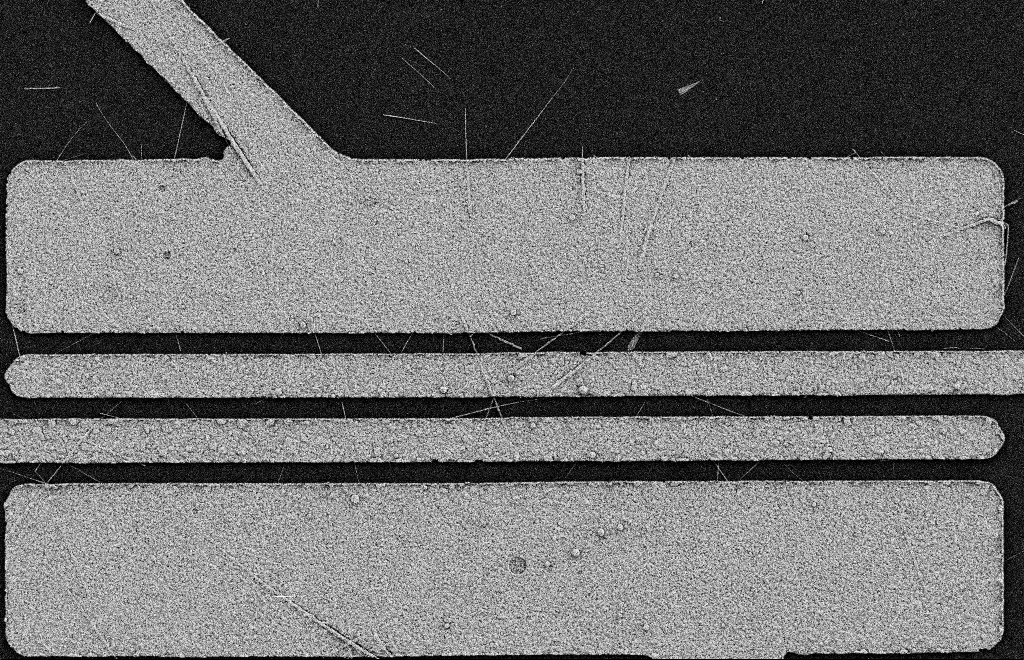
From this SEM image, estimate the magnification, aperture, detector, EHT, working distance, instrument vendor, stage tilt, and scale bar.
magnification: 5.94 K X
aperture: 20 µm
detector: SE2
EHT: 2 kV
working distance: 11 mm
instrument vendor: Zeiss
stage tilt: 0°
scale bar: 2000 nm